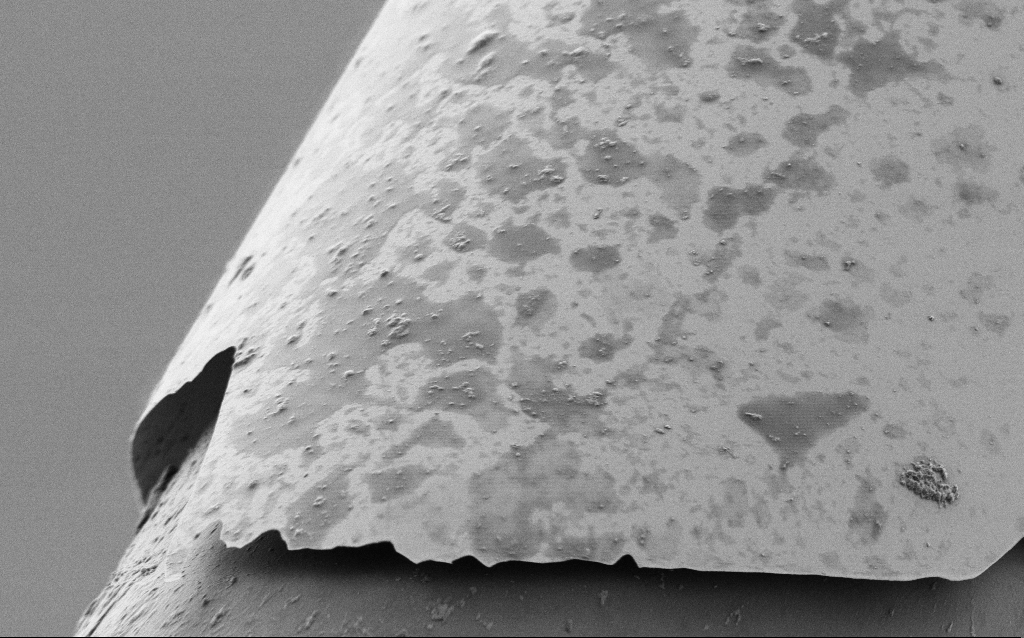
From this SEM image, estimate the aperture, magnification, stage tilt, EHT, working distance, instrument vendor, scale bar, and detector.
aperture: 30 µm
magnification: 10 K X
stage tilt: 45°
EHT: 1 kV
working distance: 7.7 mm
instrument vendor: Zeiss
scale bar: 2000 nm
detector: SE2